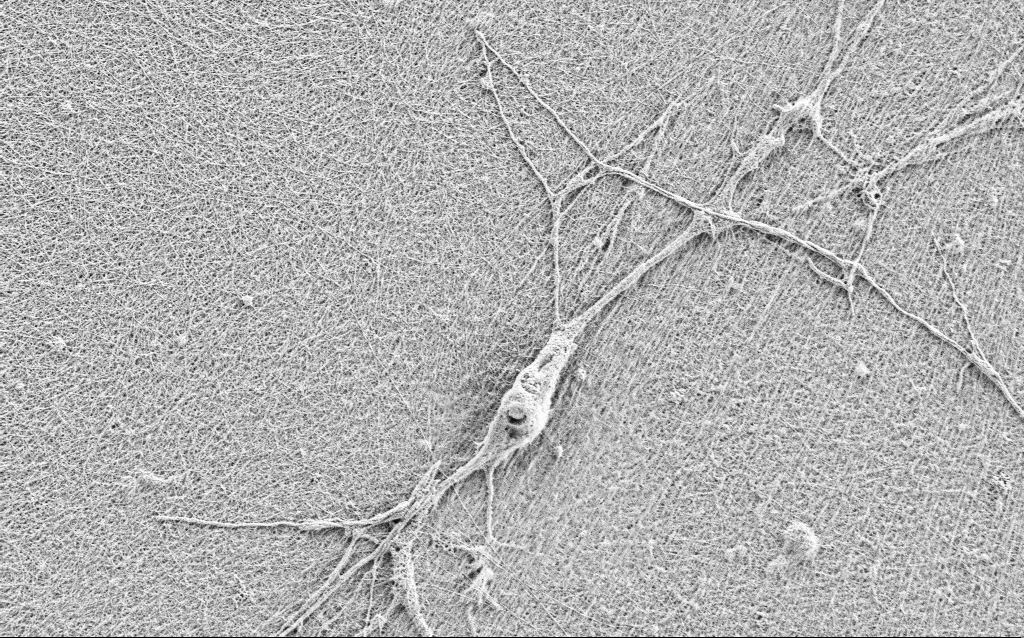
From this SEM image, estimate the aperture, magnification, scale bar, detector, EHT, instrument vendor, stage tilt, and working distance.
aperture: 30 µm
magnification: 10 K X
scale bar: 2000 nm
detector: SE2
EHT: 0.9 kV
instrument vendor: Zeiss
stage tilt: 0°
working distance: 3 mm